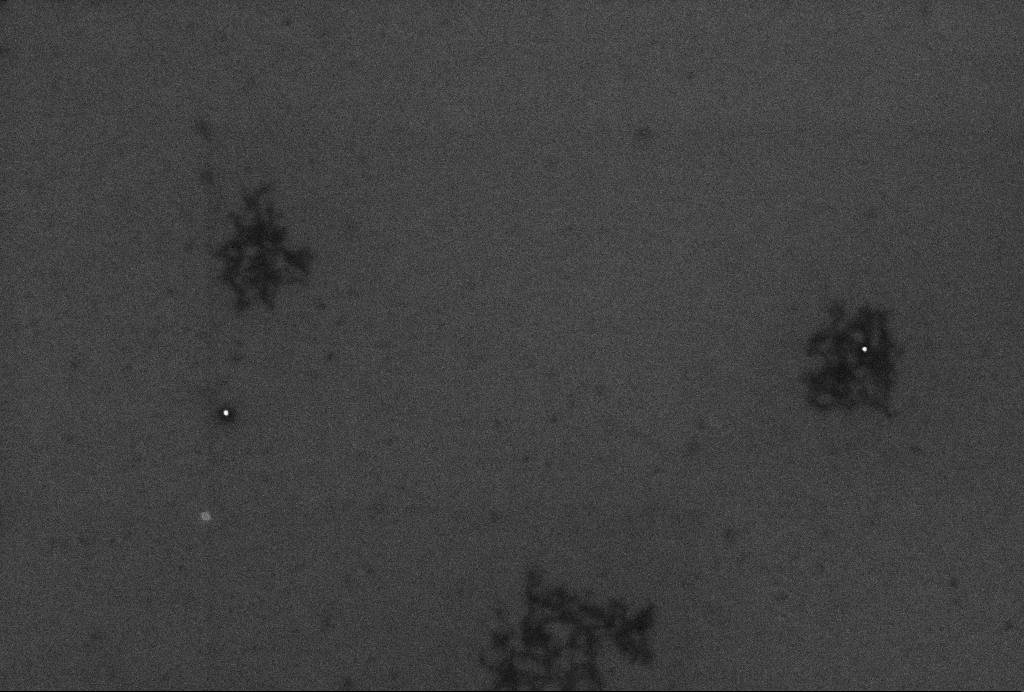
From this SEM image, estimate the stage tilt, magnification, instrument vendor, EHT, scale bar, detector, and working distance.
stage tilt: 0°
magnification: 62.65 K X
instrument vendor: Zeiss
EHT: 2 kV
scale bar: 200 nm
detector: InLens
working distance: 3.3 mm